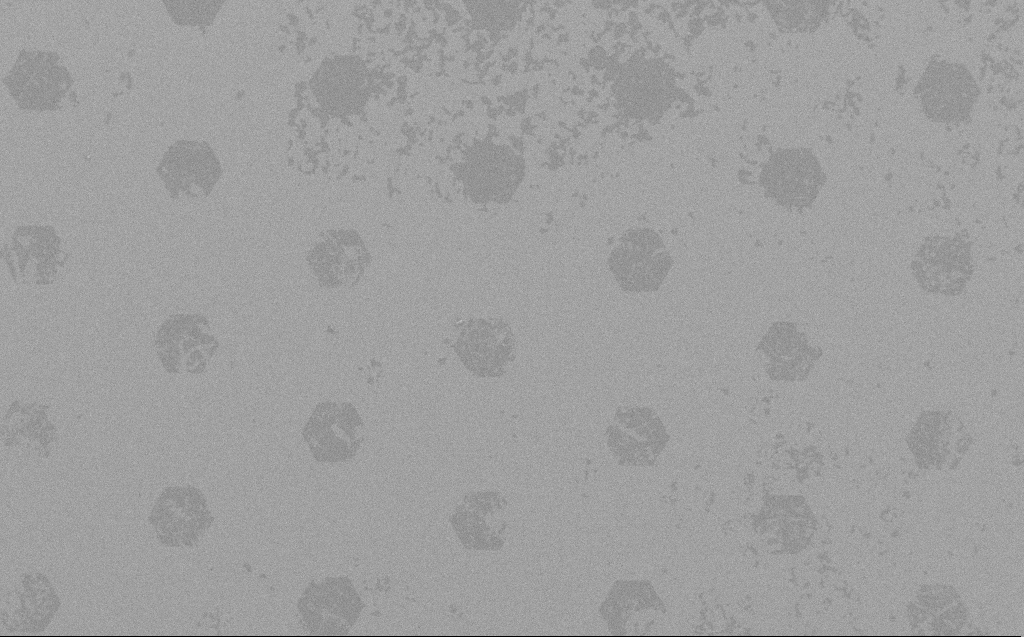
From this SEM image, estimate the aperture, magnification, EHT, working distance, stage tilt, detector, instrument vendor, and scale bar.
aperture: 30 µm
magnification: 0.432 K X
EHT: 3 kV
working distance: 5 mm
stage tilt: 0°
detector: SE2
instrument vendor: Zeiss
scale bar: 100000 nm